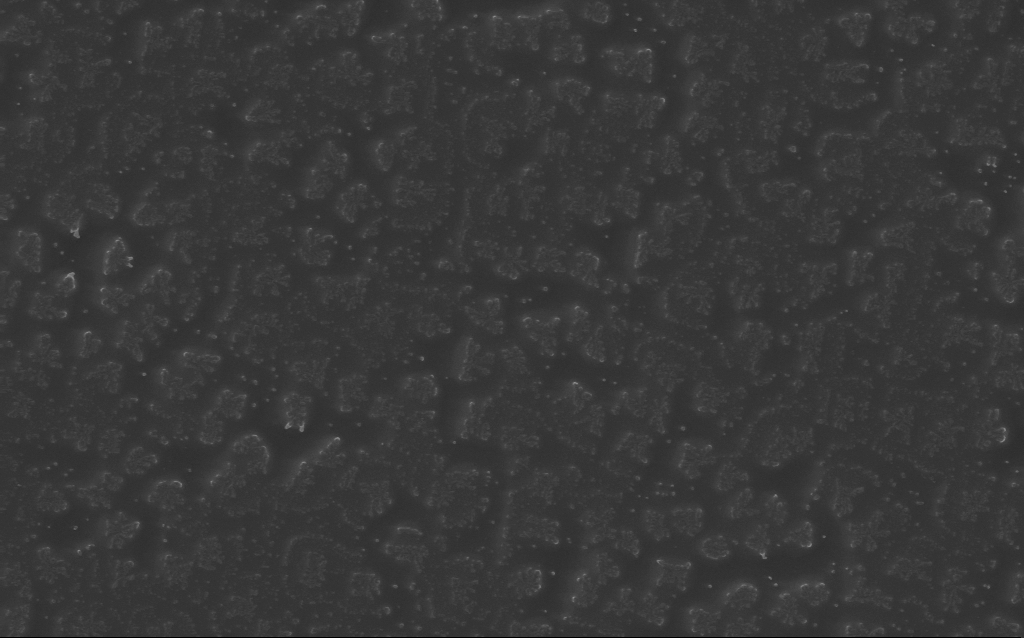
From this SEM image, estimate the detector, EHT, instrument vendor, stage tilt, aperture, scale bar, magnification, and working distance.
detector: InLens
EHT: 10 kV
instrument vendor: Zeiss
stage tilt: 0°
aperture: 30 µm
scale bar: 1000 nm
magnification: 54.84 K X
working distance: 5 mm